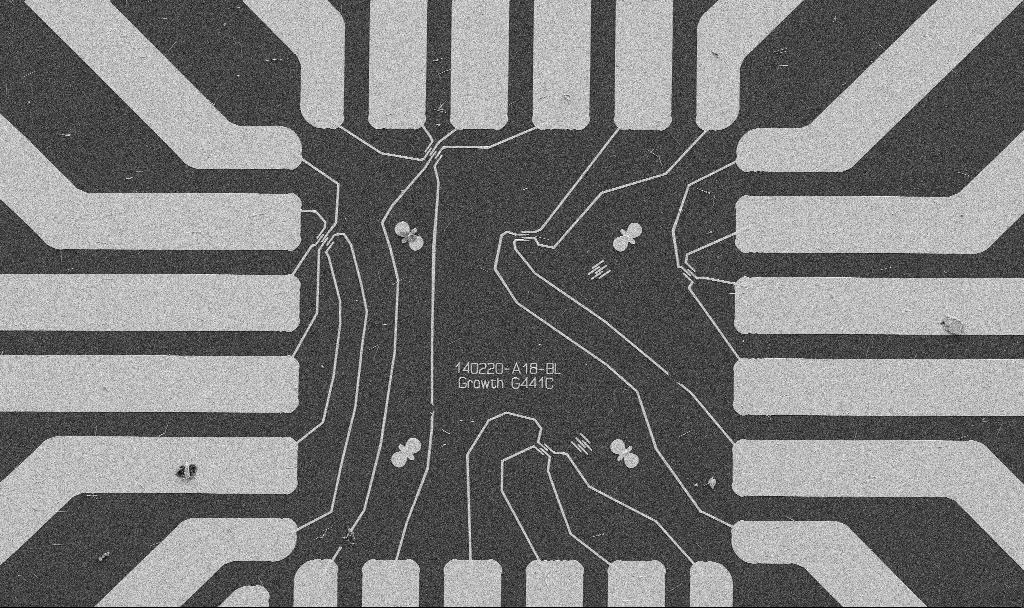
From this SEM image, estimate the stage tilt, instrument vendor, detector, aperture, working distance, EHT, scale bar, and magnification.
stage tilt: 0°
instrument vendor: Zeiss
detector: SE2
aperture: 30 µm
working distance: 10.7 mm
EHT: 5 kV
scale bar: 20000 nm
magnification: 1 K X